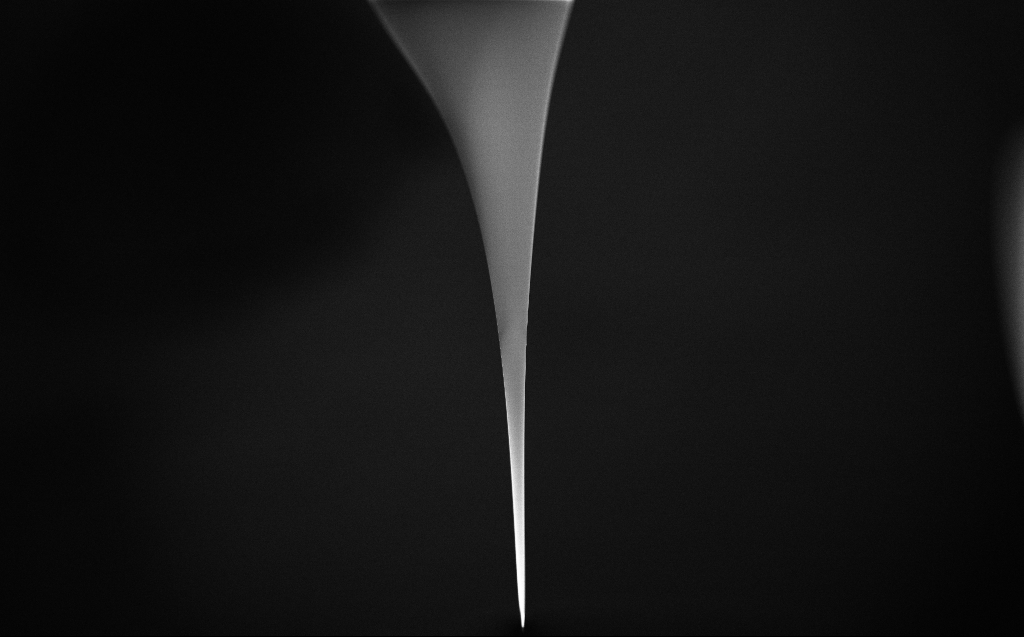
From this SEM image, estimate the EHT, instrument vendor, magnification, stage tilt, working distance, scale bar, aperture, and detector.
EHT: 2 kV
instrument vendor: Zeiss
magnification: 0.1 K X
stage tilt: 45°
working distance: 6 mm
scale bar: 200000 nm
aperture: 30 µm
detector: InLens